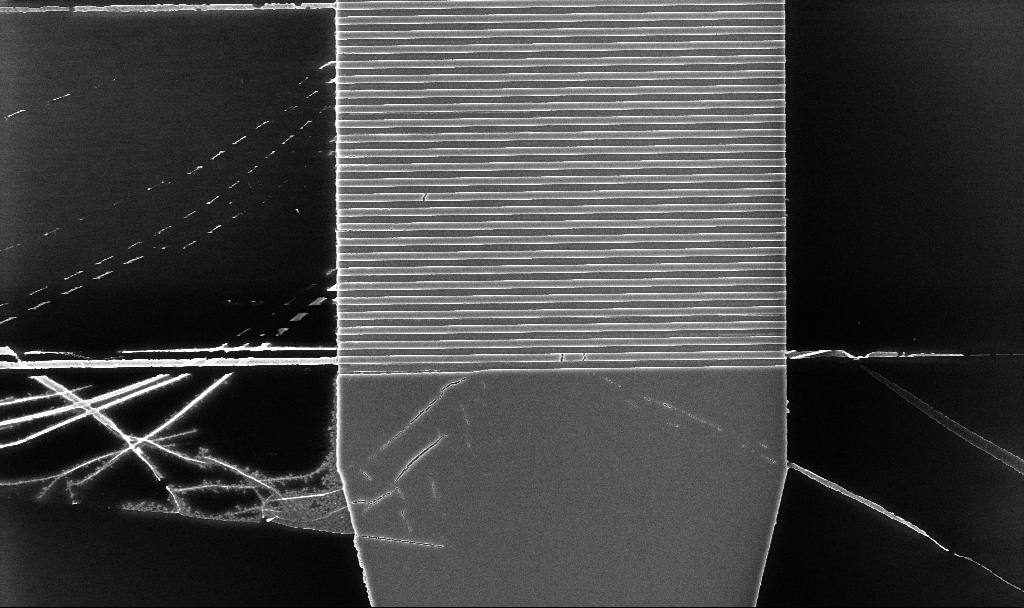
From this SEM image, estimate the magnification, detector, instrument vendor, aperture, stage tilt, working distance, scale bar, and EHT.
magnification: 8.33 K X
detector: InLens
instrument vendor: Zeiss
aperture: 30 µm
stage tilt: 0°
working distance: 5.2 mm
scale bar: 2000 nm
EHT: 5 kV